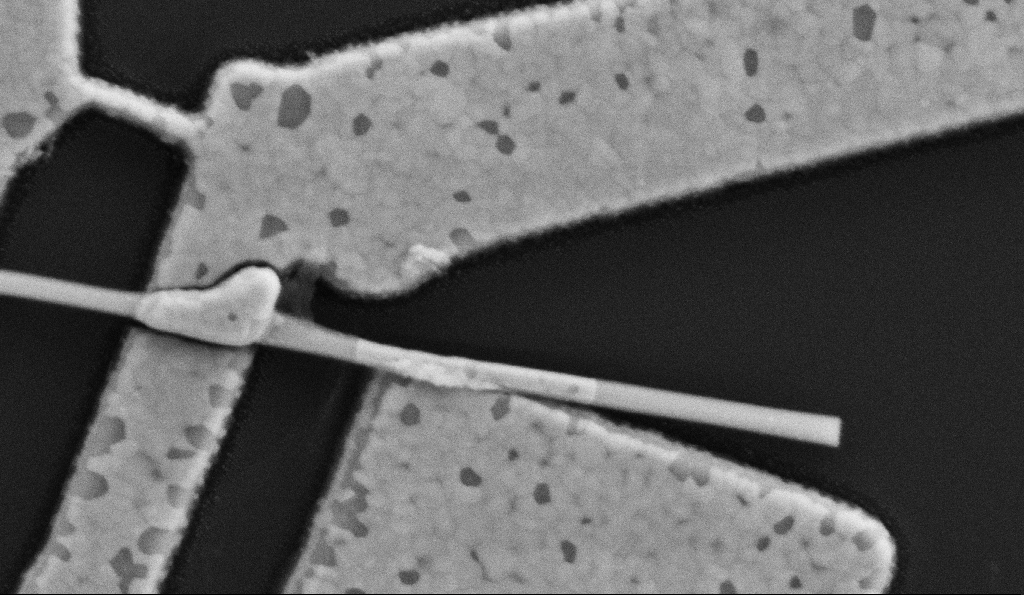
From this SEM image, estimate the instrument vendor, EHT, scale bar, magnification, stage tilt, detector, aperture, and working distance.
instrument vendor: Zeiss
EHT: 5 kV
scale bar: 200 nm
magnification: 100 K X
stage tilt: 0°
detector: SE2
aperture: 30 µm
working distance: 9.5 mm